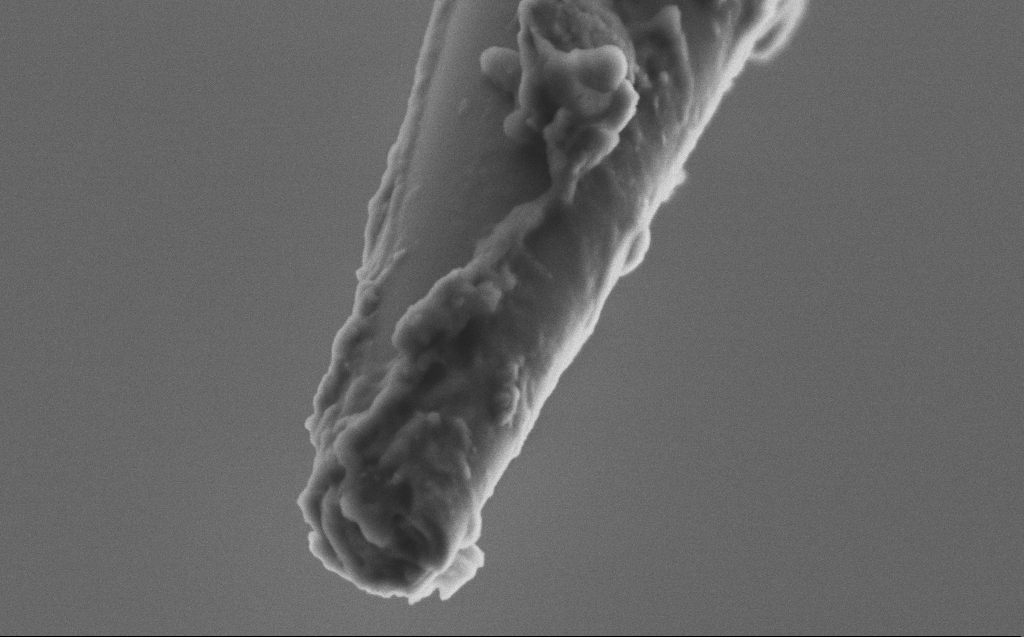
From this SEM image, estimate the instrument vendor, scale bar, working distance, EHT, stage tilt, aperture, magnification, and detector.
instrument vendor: Zeiss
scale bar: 200 nm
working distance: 3 mm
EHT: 2 kV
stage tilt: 45°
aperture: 30 µm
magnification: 100 K X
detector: SE2